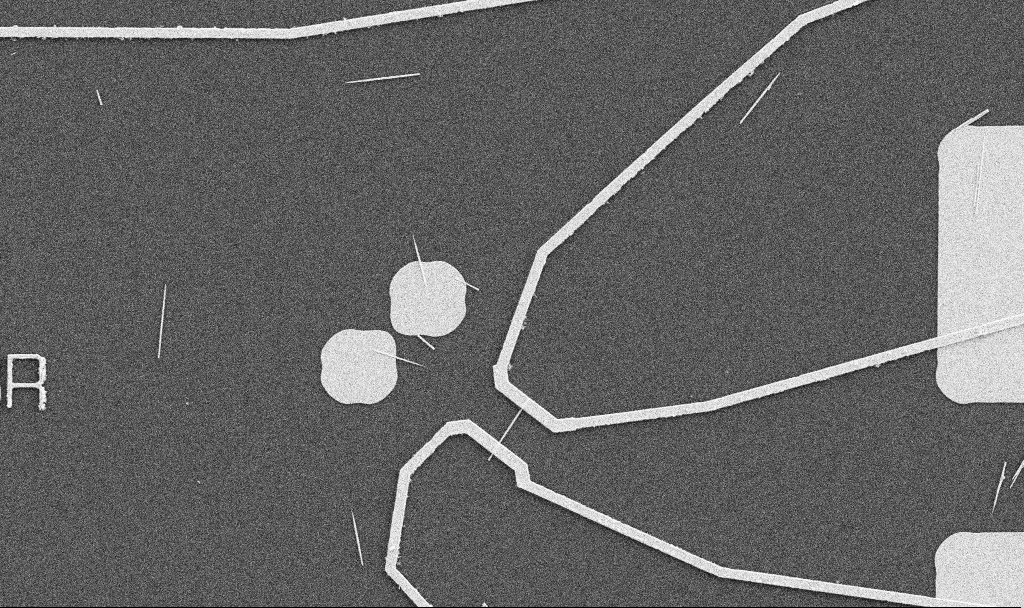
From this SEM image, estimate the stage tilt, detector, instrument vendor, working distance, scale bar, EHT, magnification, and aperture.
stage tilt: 0°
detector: SE2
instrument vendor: Zeiss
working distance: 10.7 mm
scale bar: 10000 nm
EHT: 5 kV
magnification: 5 K X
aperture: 30 µm